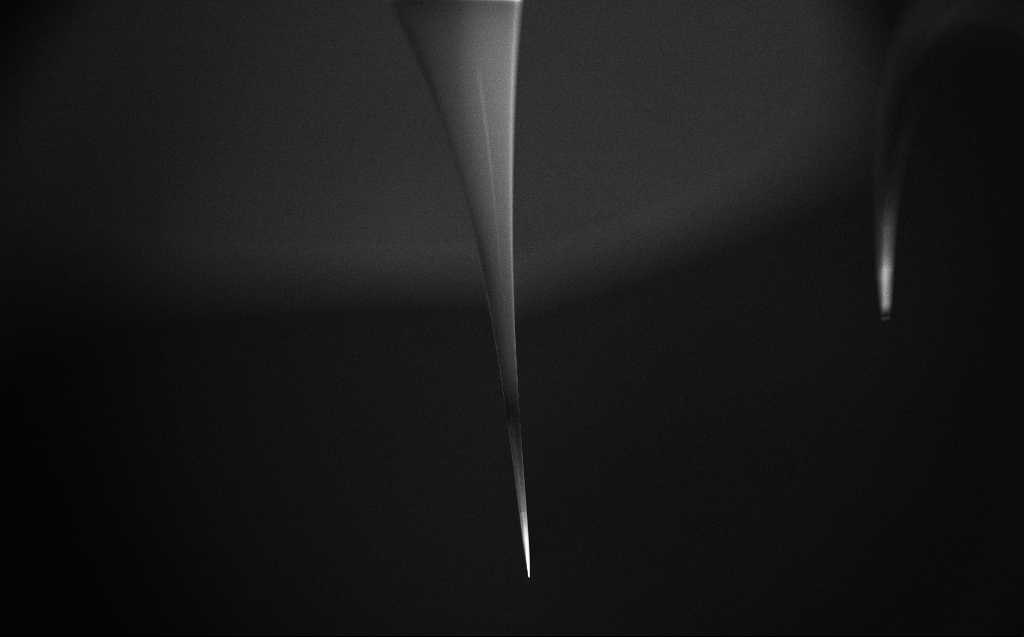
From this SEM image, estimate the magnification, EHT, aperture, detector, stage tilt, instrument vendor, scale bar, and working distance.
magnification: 0.1 K X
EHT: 2 kV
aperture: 30 µm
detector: InLens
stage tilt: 45°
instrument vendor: Zeiss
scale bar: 200000 nm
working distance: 6 mm